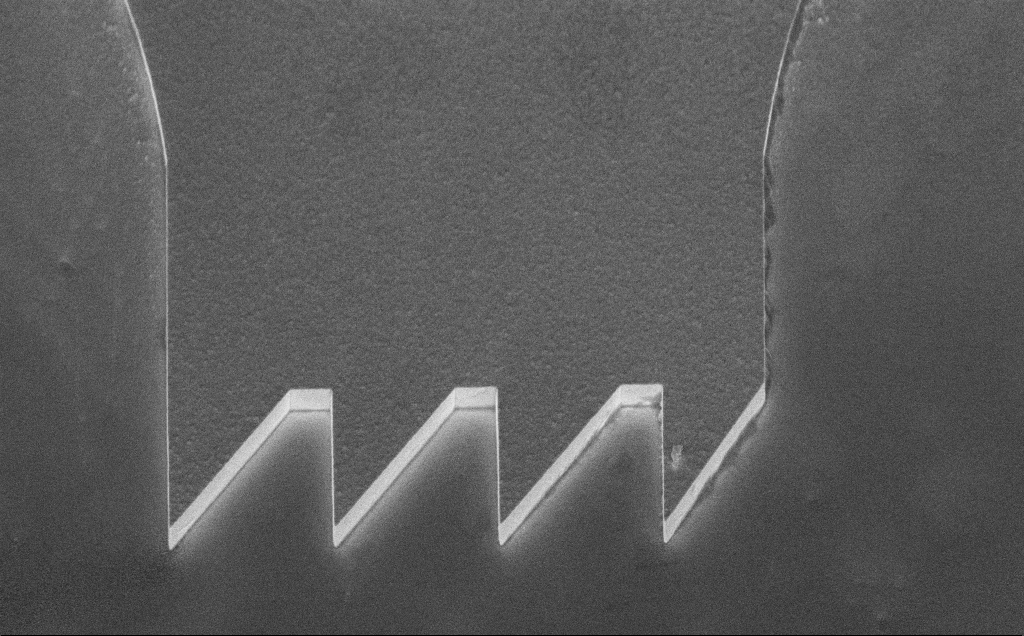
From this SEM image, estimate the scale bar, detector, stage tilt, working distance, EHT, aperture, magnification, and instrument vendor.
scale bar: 10000 nm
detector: InLens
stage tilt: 45°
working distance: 8 mm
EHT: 10 kV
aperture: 30 µm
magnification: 5.26 K X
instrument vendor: Zeiss